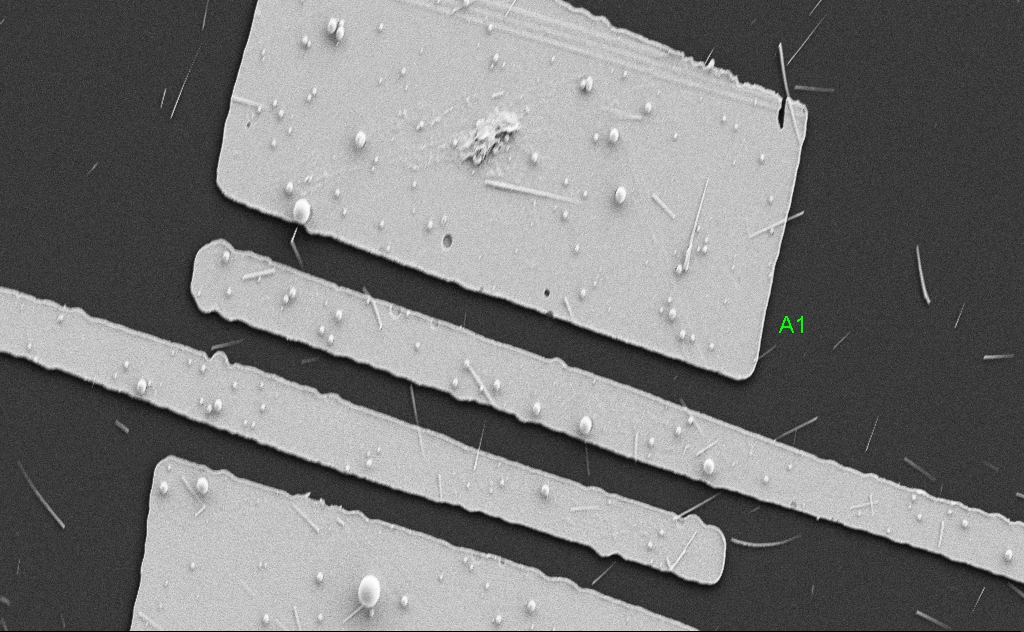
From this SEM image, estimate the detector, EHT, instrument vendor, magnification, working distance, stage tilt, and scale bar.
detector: SE2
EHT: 5 kV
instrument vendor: Zeiss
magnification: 7.18 K X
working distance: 5 mm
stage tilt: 0°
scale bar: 10000 nm